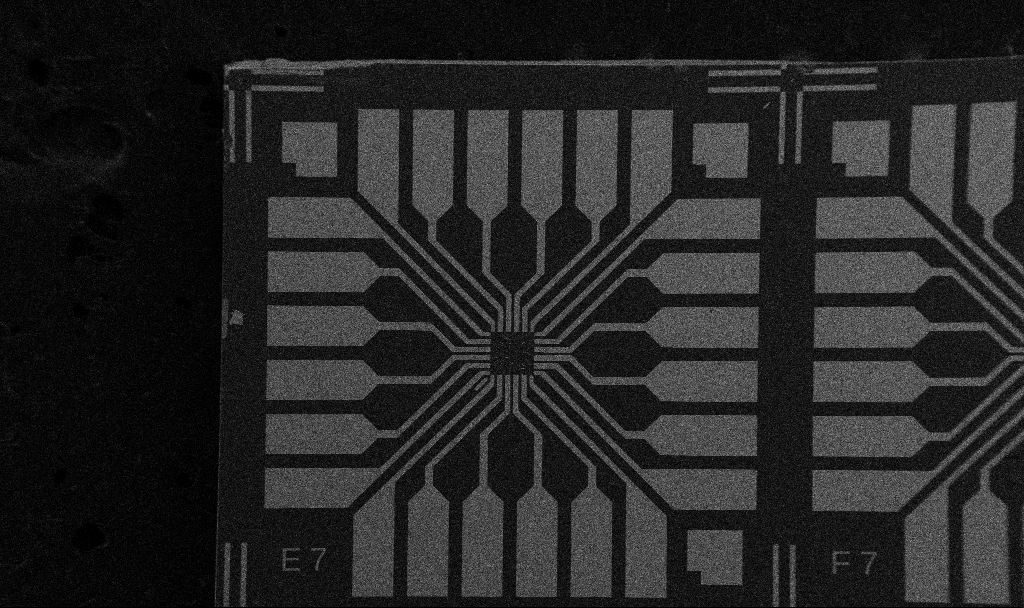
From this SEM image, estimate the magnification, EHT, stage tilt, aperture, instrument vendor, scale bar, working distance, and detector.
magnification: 0.1 K X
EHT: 5 kV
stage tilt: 0°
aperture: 30 µm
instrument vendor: Zeiss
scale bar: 200000 nm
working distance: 10.7 mm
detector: SE2